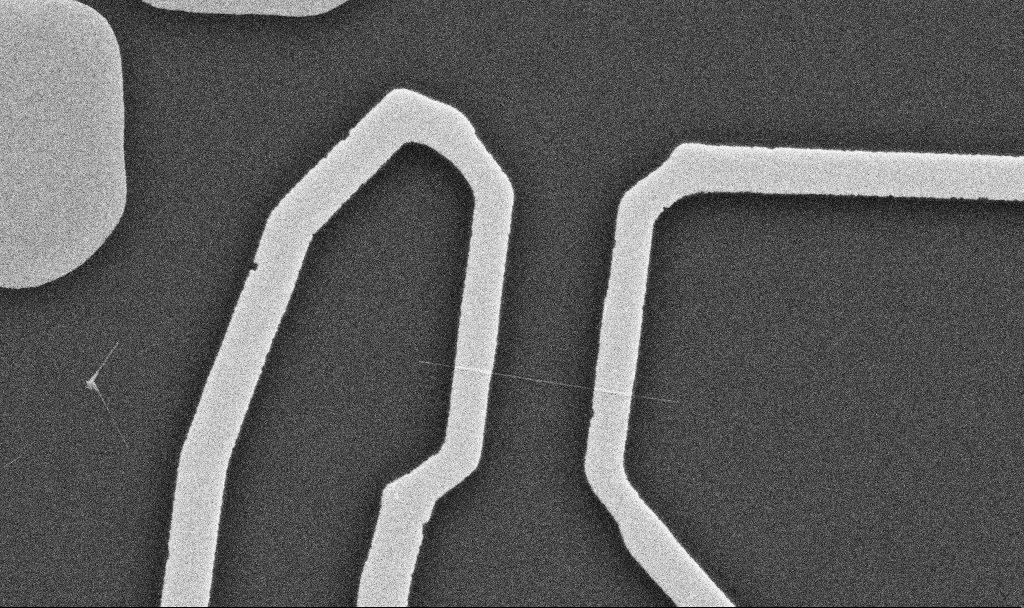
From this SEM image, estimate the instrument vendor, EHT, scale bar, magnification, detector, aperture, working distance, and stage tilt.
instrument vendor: Zeiss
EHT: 10 kV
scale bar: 1000 nm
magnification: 20 K X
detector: SE2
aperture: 30 µm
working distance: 10.7 mm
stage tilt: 0°